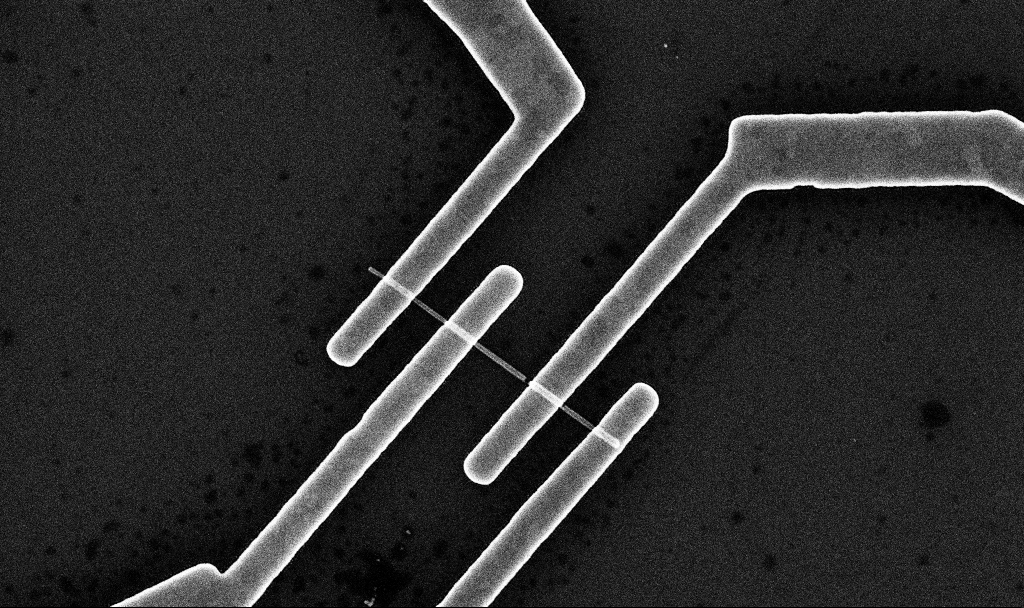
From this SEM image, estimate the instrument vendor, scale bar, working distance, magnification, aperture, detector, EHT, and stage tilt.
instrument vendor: Zeiss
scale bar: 2000 nm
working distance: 7 mm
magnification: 32.07 K X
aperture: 30 µm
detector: InLens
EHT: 10 kV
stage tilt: -0°